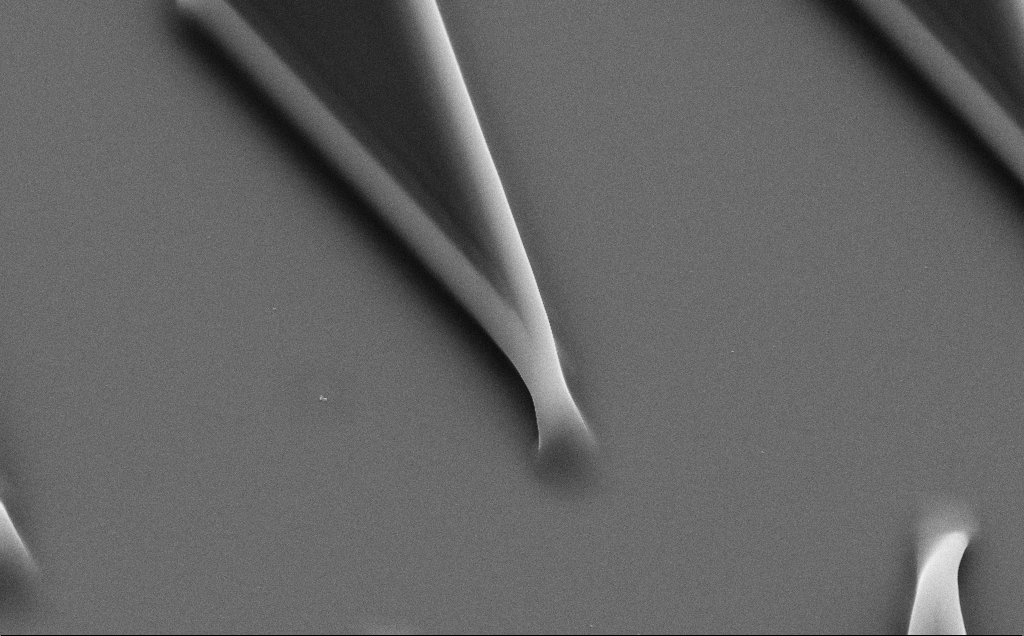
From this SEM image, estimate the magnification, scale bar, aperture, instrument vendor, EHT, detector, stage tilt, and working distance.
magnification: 12.53 K X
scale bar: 1000 nm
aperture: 30 µm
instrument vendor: Zeiss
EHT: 10 kV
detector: SE2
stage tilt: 35°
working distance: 8 mm